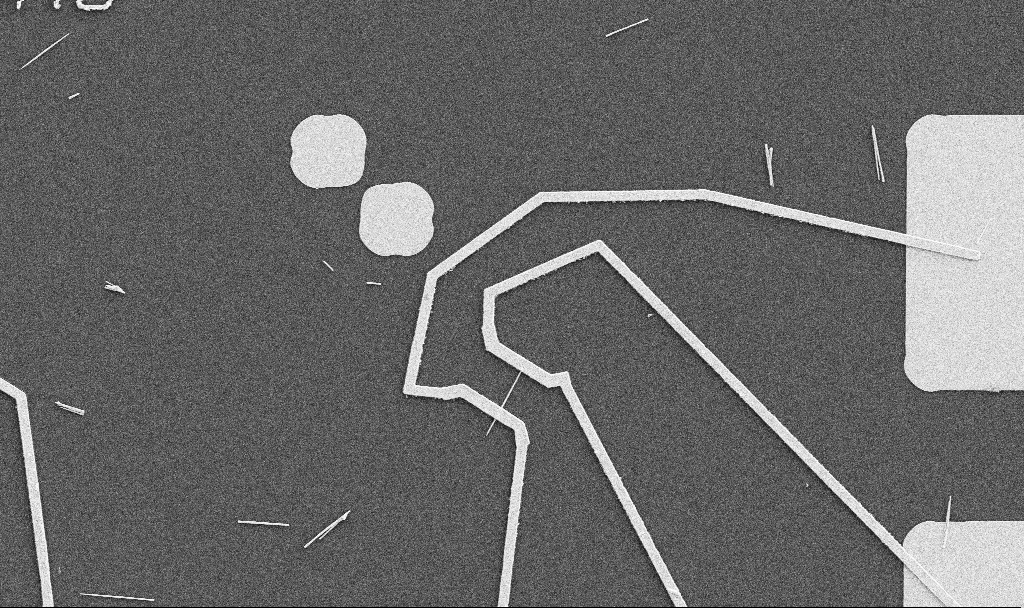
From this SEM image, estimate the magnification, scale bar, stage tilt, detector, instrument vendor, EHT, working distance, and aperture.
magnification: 5 K X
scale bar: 10000 nm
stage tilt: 0°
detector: SE2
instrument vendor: Zeiss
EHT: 5 kV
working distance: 10.7 mm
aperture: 30 µm